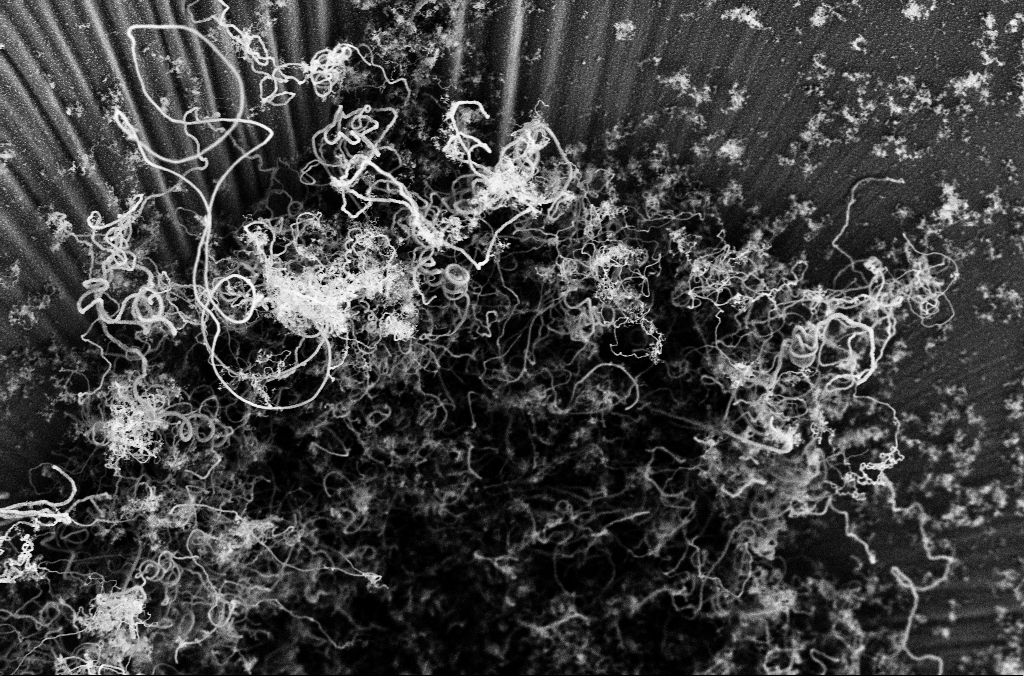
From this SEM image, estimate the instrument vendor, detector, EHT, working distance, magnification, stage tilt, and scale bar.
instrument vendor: Zeiss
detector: SE2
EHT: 3 kV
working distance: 5.1 mm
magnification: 10 K X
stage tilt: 0°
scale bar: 2000 nm